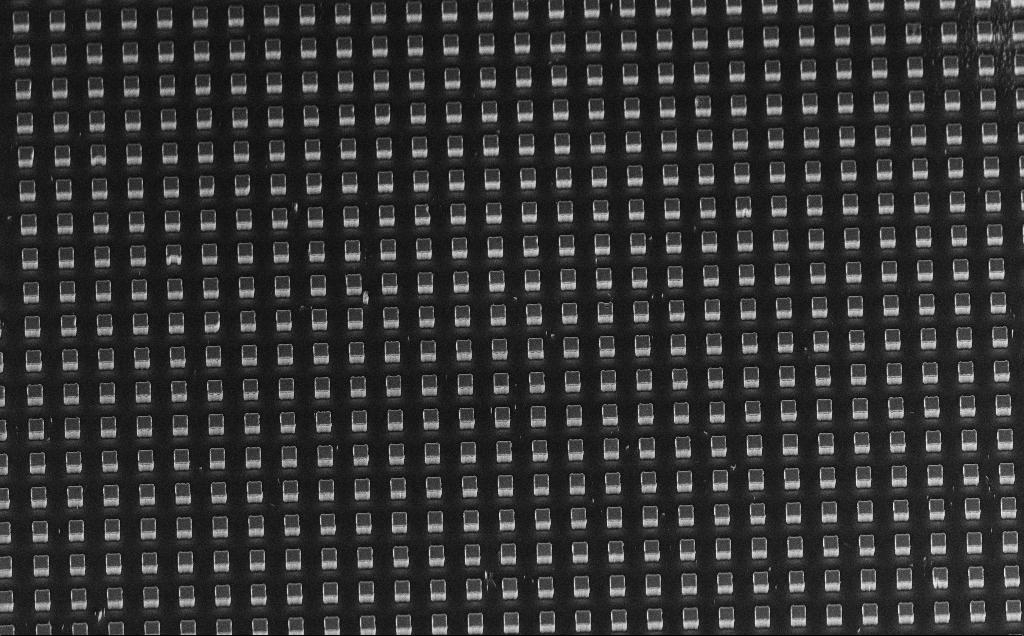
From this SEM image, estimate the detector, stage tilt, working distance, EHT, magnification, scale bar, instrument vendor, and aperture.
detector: InLens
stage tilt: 45°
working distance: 11 mm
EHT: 10 kV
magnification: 0.659 K X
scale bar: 100000 nm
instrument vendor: Zeiss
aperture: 30 µm